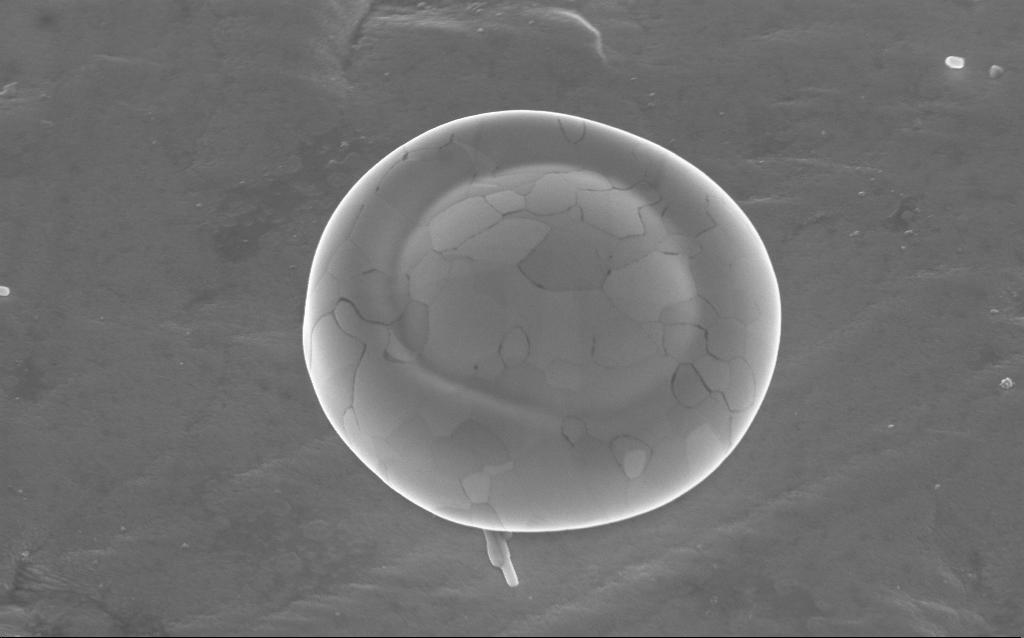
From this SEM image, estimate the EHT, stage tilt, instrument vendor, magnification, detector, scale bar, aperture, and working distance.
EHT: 5 kV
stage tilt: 35°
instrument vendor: Zeiss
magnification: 41.93 K X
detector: InLens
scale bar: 1000 nm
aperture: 30 µm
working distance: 4 mm